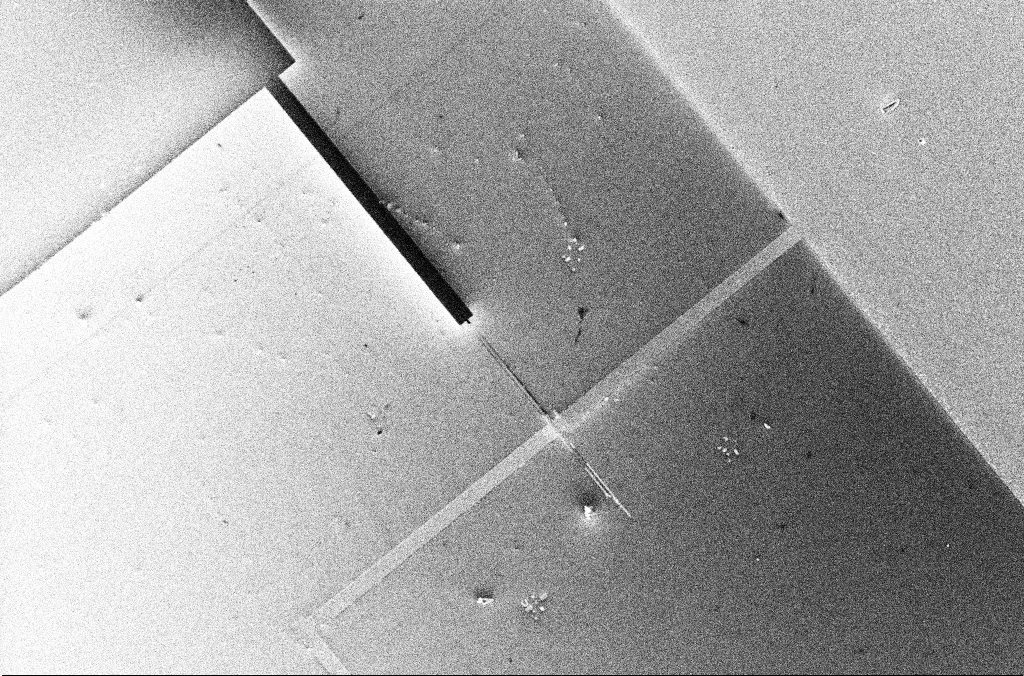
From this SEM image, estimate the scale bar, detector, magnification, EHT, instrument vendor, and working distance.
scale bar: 20000 nm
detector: InLens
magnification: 1.15 K X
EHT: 5 kV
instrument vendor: Zeiss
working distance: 3.4 mm